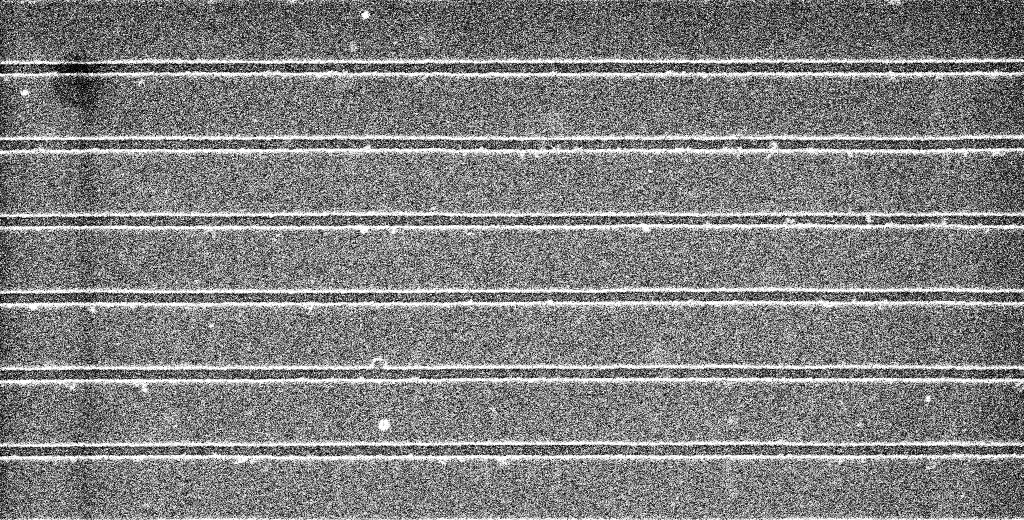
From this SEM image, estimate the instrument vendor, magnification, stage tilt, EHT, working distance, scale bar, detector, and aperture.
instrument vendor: Zeiss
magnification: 31.33 K X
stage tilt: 0°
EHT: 5 kV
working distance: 5.3 mm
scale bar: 1000 nm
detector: InLens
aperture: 30 µm